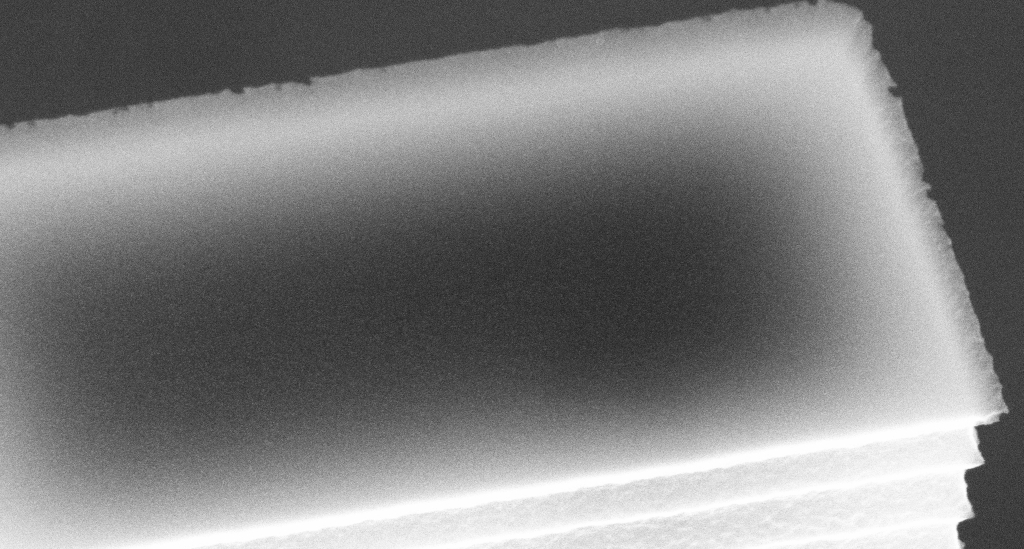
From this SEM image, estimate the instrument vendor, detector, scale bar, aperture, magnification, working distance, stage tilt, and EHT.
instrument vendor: Zeiss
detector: InLens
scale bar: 200 nm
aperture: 30 µm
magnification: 81.35 K X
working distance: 7 mm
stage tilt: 45°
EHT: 10 kV